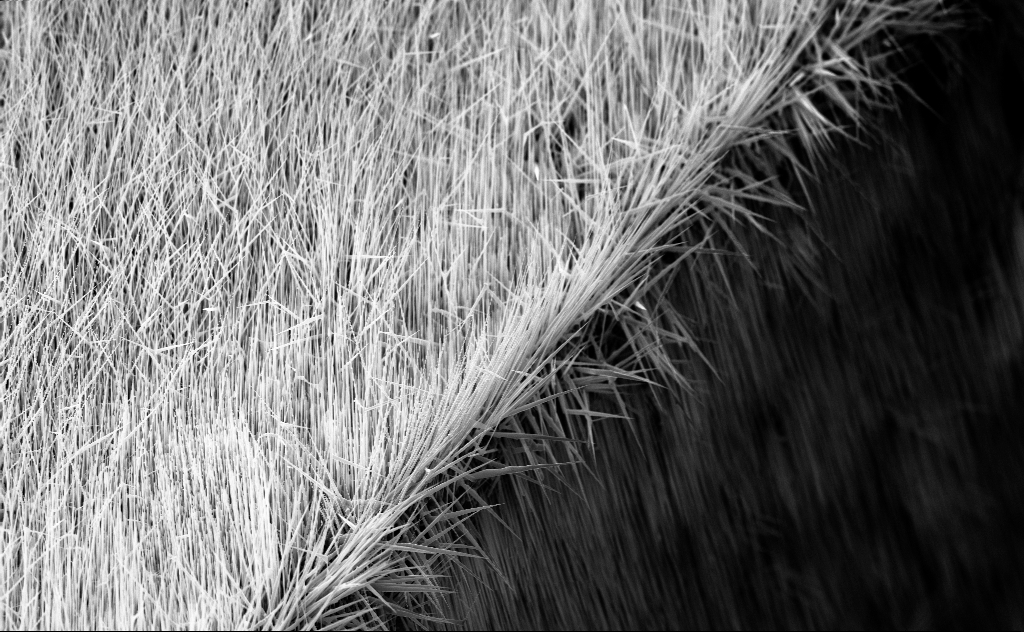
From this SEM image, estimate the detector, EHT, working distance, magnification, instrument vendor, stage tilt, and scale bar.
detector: InLens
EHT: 10 kV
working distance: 6 mm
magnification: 3.64 K X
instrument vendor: Zeiss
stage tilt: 45°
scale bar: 10000 nm